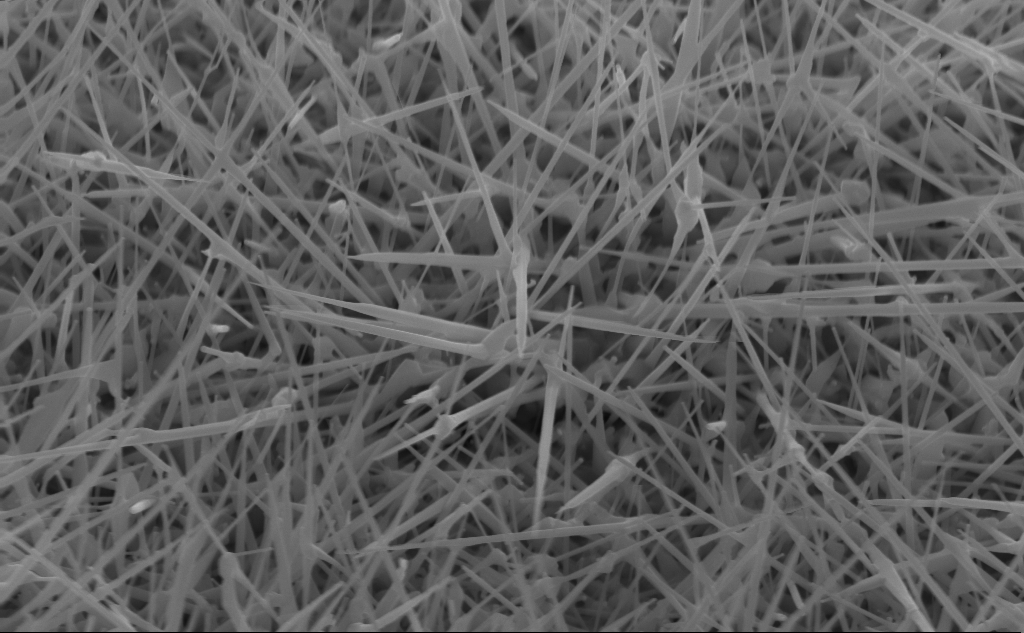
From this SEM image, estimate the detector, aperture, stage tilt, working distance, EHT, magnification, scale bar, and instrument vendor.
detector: InLens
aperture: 30 µm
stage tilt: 45°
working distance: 4 mm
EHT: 10 kV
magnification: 40 K X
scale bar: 1000 nm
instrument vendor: Zeiss